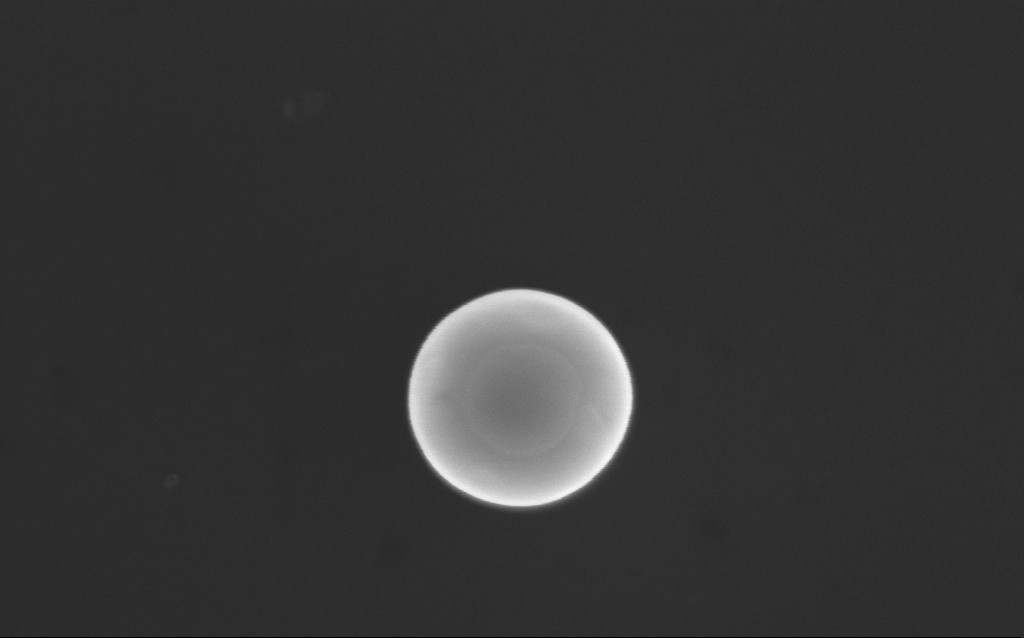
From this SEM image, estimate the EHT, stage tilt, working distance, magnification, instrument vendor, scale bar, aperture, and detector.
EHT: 10 kV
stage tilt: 0°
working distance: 4 mm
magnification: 132.49 K X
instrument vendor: Zeiss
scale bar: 200 nm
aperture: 30 µm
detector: InLens